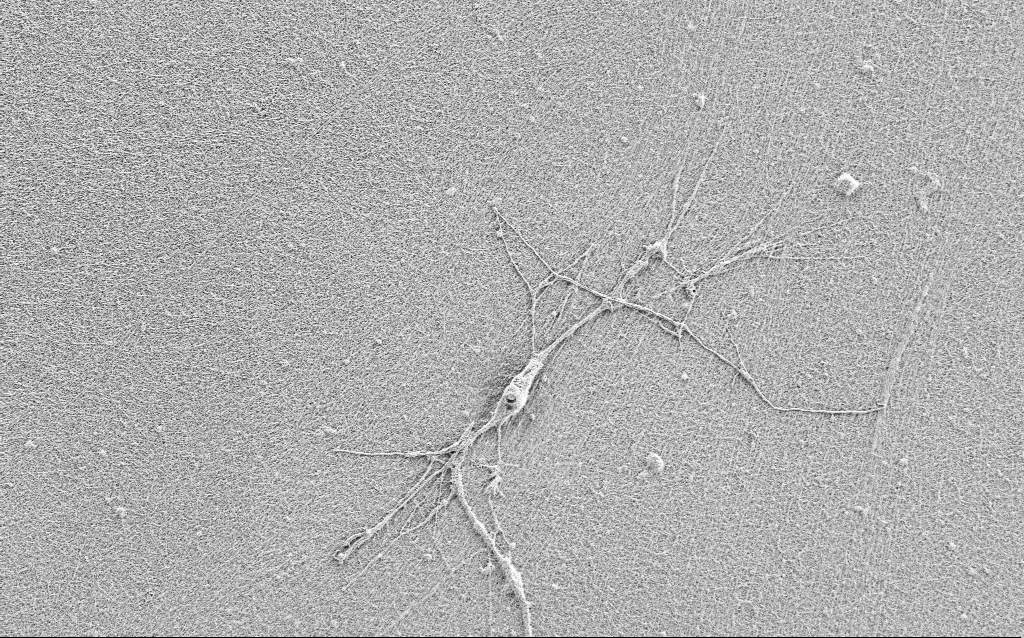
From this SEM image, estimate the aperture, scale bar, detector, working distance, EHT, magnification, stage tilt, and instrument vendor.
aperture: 30 µm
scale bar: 10000 nm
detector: SE2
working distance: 3 mm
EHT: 0.9 kV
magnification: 5 K X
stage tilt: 0°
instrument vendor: Zeiss